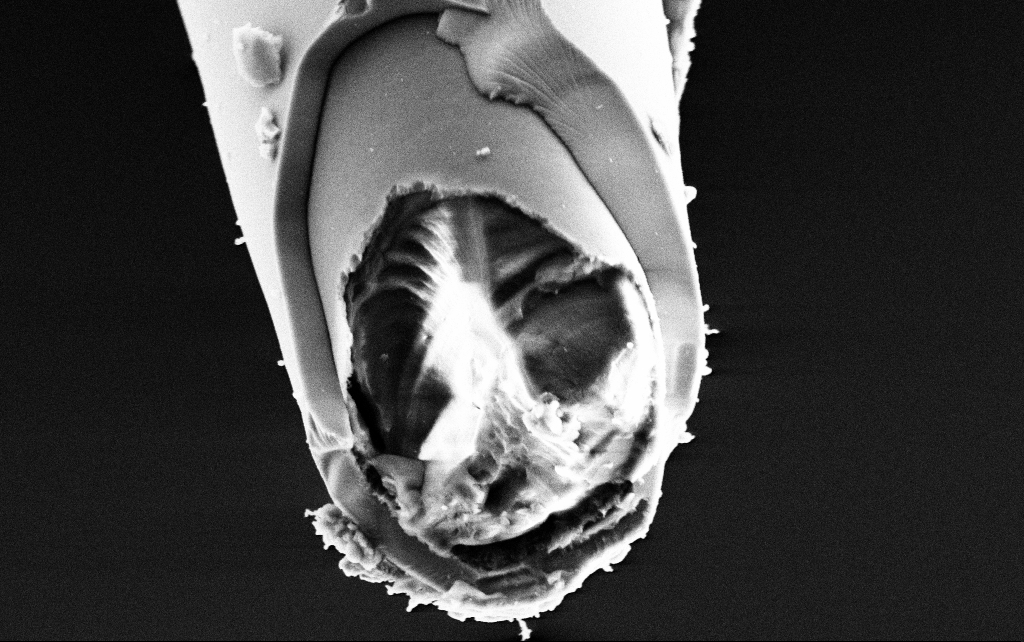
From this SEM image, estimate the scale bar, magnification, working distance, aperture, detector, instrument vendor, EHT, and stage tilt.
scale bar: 2000 nm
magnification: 25 K X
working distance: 7.9 mm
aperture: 30 µm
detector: SE2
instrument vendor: Zeiss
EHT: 3 kV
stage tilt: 45°